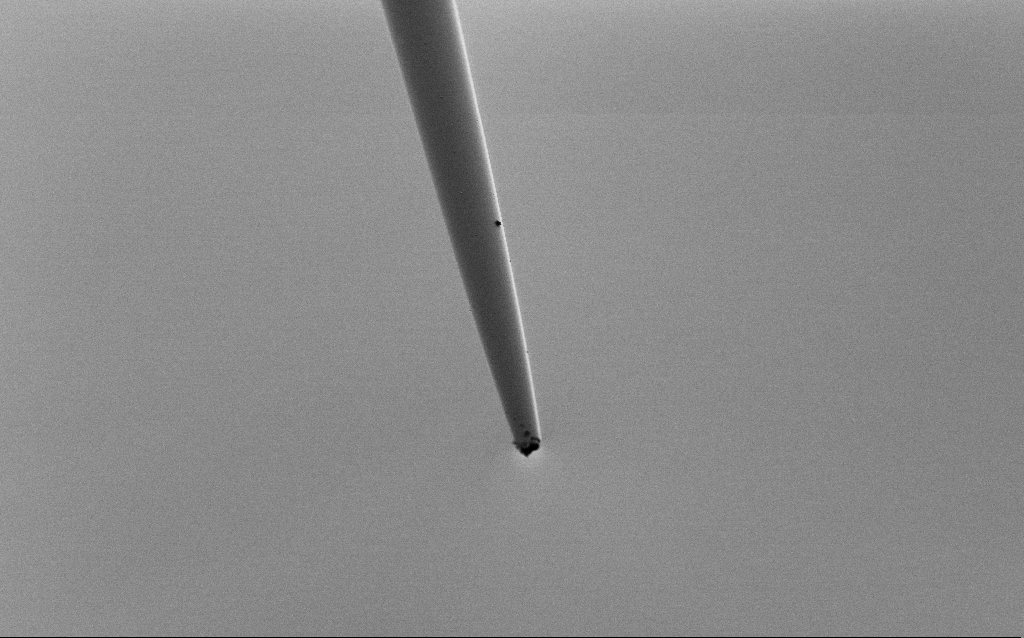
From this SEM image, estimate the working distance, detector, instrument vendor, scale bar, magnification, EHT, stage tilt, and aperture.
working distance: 6 mm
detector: SE2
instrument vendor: Zeiss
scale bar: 20000 nm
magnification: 1 K X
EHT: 2 kV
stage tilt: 45°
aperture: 30 µm